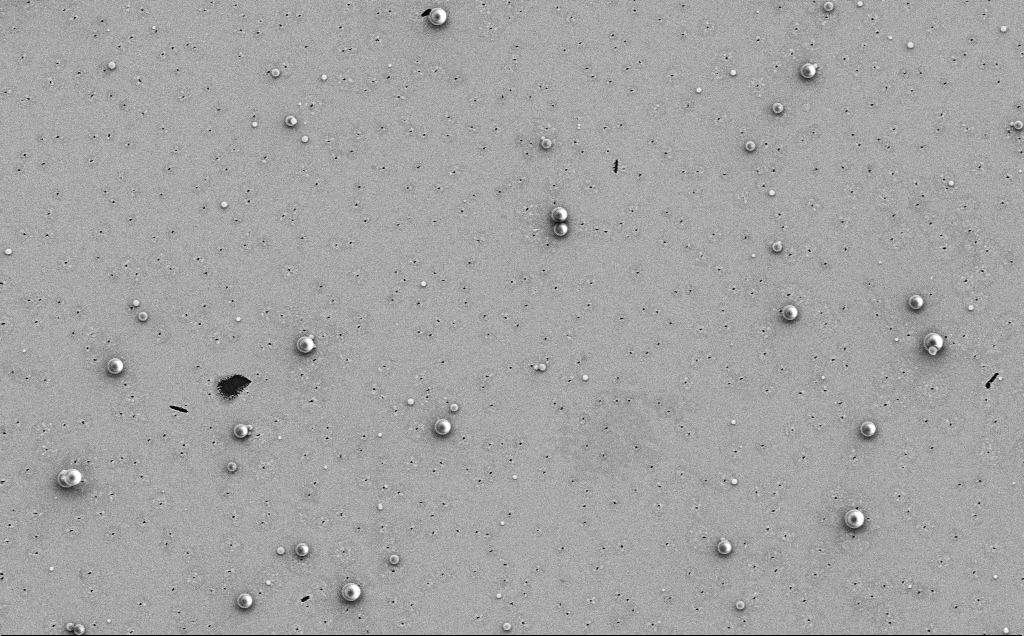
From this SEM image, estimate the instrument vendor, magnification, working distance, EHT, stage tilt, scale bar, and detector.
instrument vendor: Zeiss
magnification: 4.2 K X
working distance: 12 mm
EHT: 5 kV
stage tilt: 0°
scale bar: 10000 nm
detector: SE2